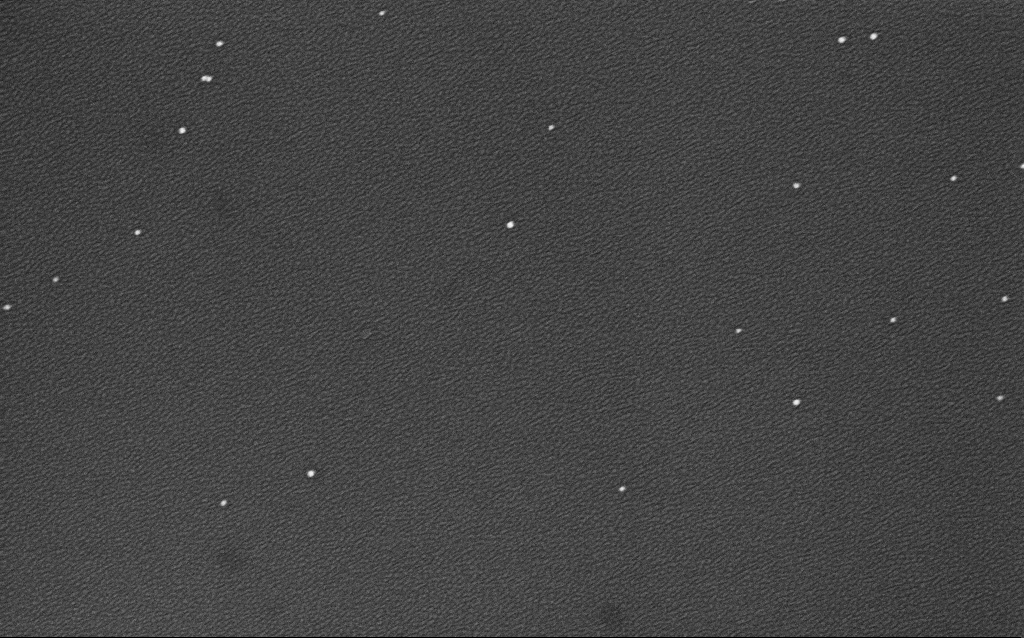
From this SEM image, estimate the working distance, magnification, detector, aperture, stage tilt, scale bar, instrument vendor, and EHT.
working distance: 2.1 mm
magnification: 100 K X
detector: InLens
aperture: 30 µm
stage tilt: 0°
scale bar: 200 nm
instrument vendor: Zeiss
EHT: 4 kV